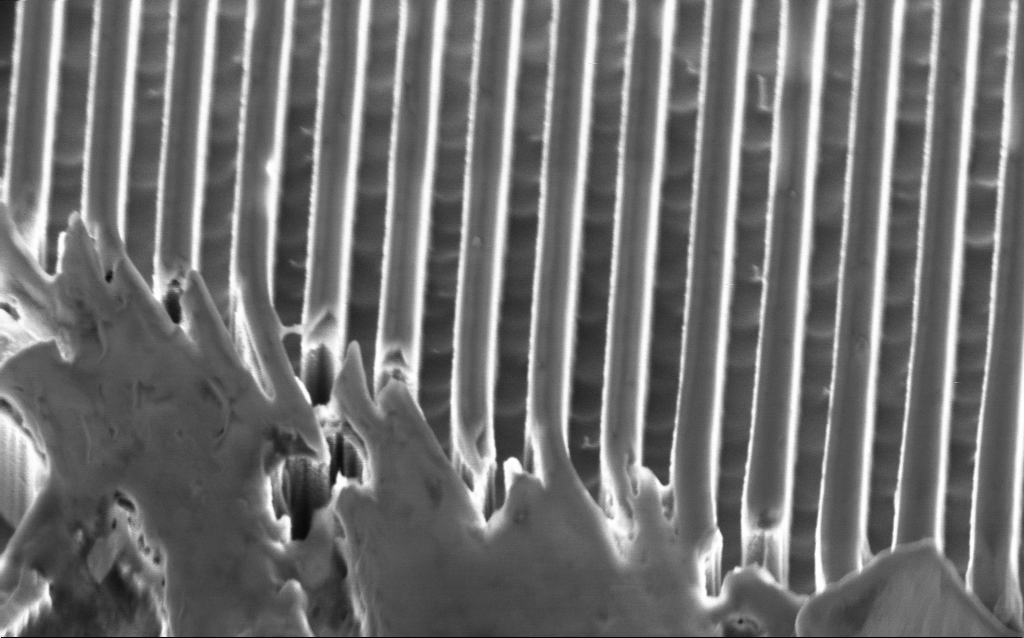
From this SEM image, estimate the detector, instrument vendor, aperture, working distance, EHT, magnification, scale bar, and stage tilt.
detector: InLens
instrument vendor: Zeiss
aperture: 30 µm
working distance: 3.3 mm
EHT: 2 kV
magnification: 56.34 K X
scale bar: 1000 nm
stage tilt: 45°